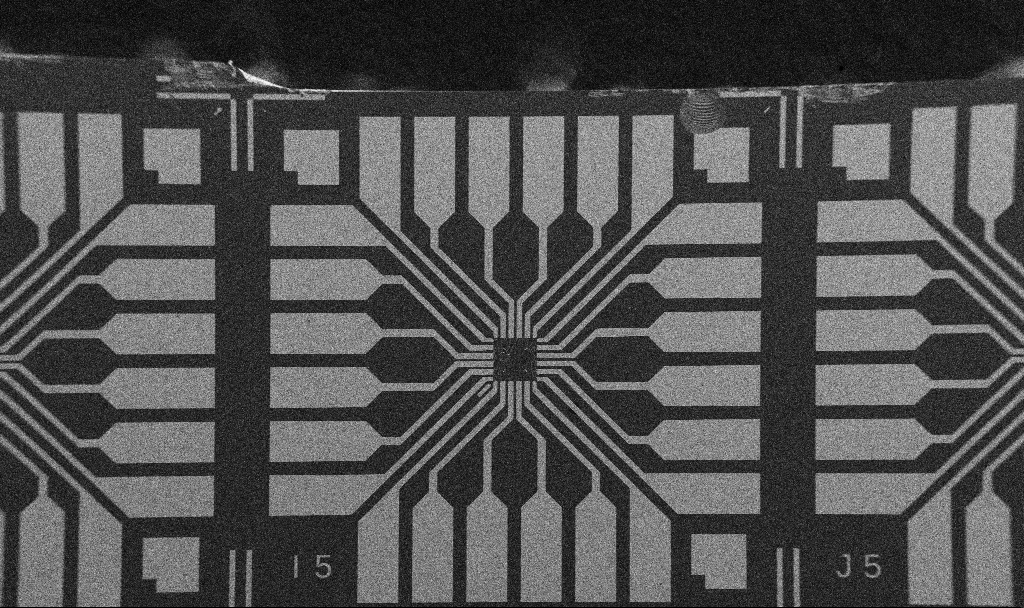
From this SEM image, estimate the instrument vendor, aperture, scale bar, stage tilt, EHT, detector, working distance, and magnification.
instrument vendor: Zeiss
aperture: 30 µm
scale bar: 200000 nm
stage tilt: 0°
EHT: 5 kV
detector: SE2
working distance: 10.7 mm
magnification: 0.1 K X